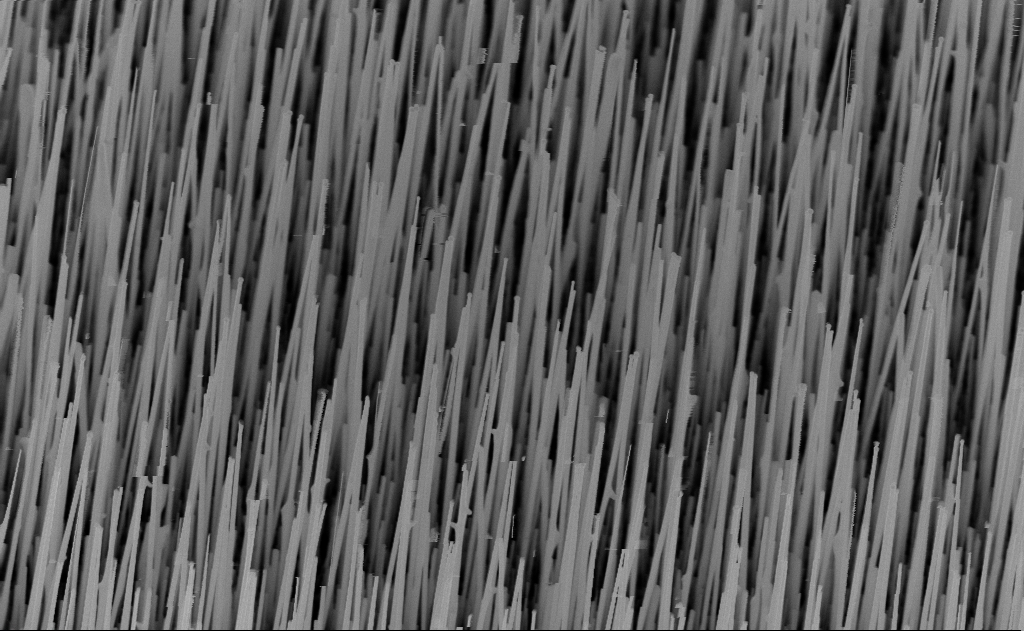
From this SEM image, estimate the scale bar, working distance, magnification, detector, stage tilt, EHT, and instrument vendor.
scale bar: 1000 nm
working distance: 9 mm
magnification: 20 K X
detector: InLens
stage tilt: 30°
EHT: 10 kV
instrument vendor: Zeiss